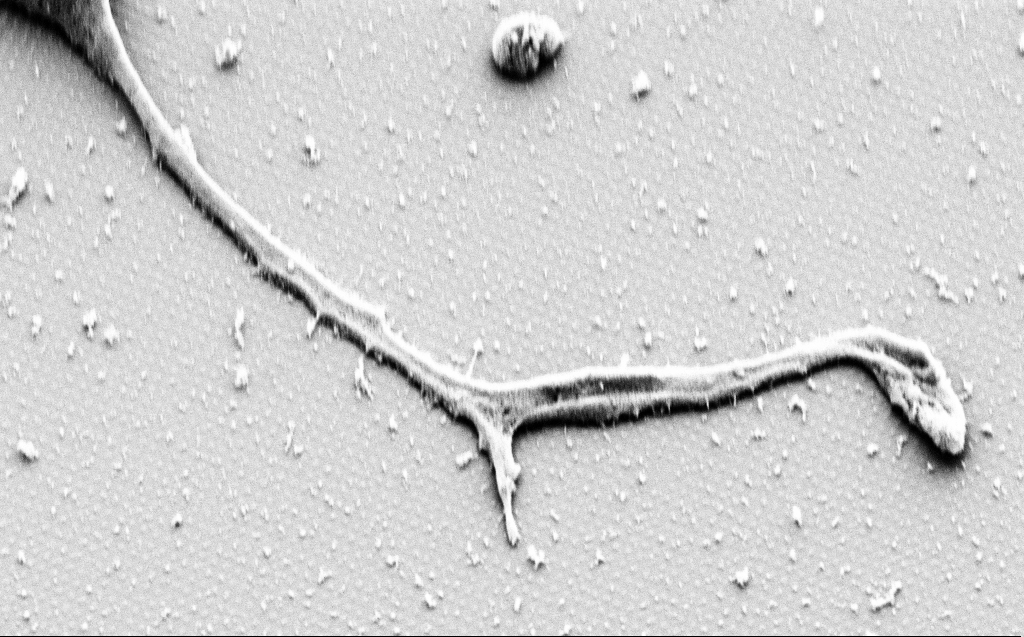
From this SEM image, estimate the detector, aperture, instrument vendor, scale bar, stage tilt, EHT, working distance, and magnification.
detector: SE2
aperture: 30 µm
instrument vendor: Zeiss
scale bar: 2000 nm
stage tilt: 45°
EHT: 3 kV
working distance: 9 mm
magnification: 9.55 K X